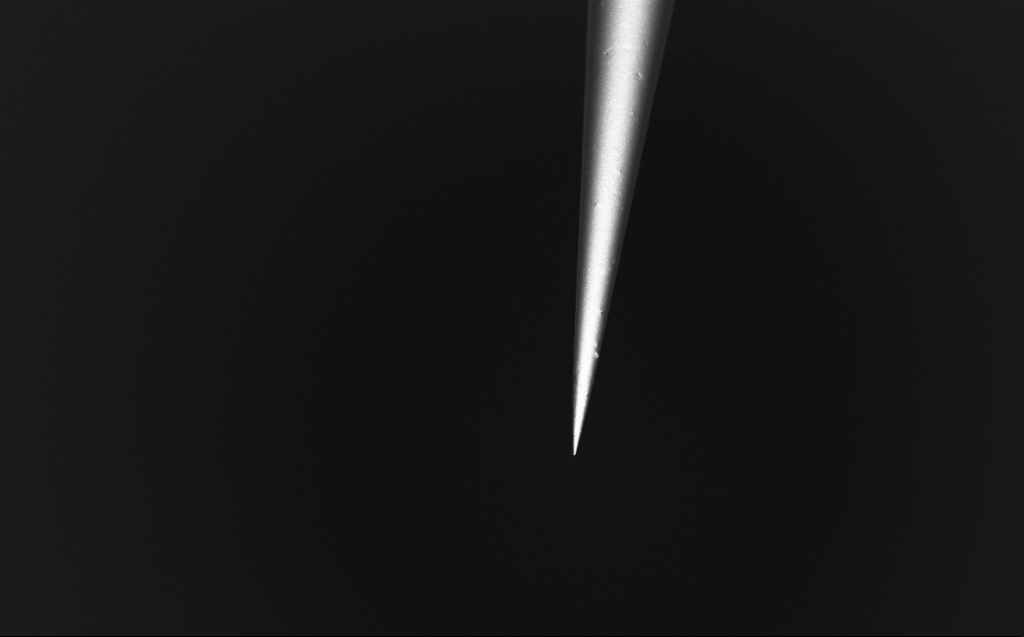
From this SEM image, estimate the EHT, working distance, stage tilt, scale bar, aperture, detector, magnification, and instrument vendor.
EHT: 1 kV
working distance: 5 mm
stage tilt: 45°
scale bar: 10000 nm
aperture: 30 µm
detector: InLens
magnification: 5 K X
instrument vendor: Zeiss